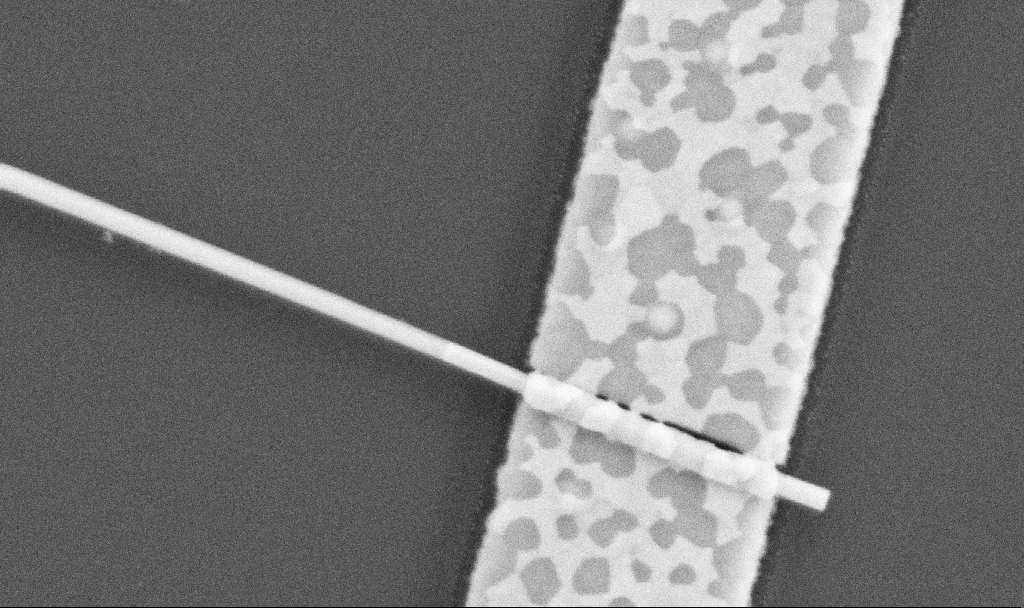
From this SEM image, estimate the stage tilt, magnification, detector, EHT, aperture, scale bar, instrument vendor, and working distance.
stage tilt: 0°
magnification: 100 K X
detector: SE2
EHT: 5 kV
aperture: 30 µm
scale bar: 200 nm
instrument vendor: Zeiss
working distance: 10.5 mm